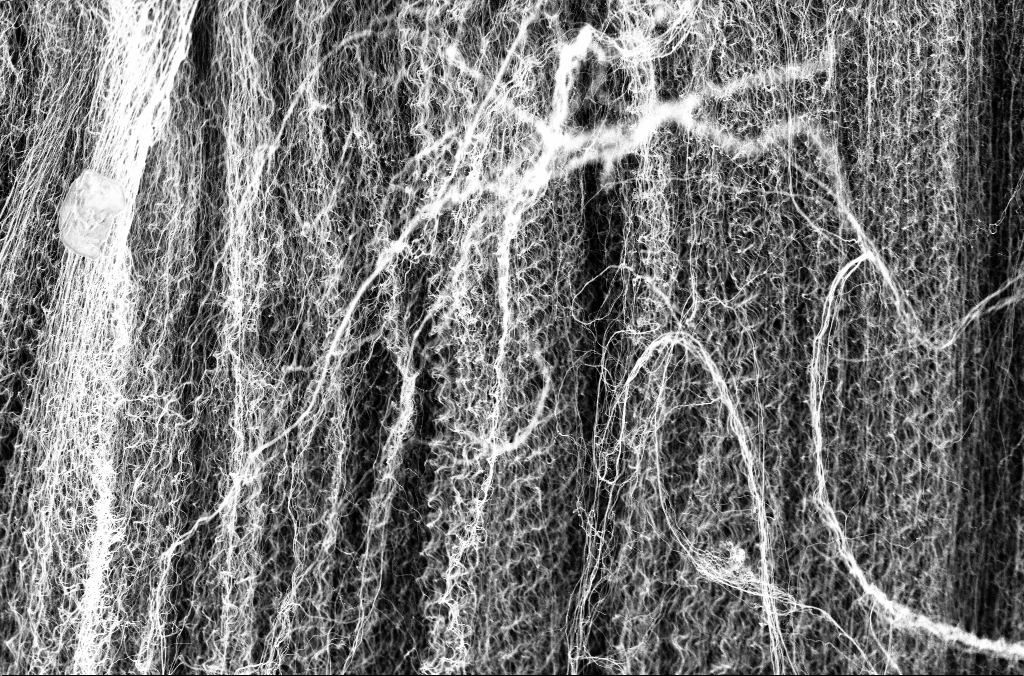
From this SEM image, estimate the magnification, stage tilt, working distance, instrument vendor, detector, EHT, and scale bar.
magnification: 10 K X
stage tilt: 45°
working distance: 3.3 mm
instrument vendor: Zeiss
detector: InLens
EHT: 3 kV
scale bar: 2000 nm